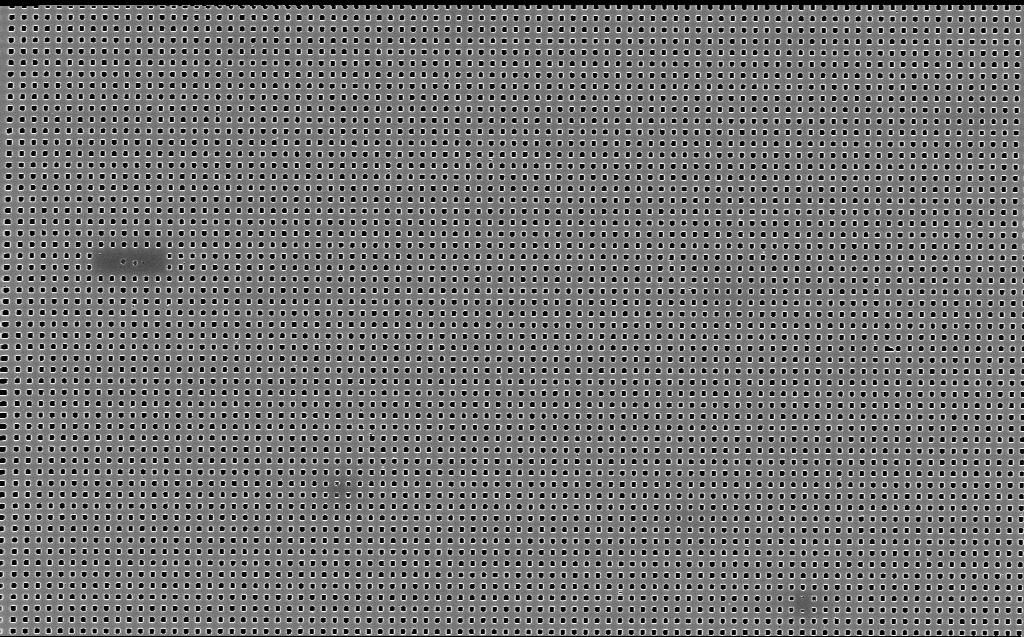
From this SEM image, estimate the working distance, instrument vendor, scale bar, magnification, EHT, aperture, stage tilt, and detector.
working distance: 6 mm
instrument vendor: Zeiss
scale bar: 2000 nm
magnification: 8.48 K X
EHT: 10 kV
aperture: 30 µm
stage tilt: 0°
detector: InLens